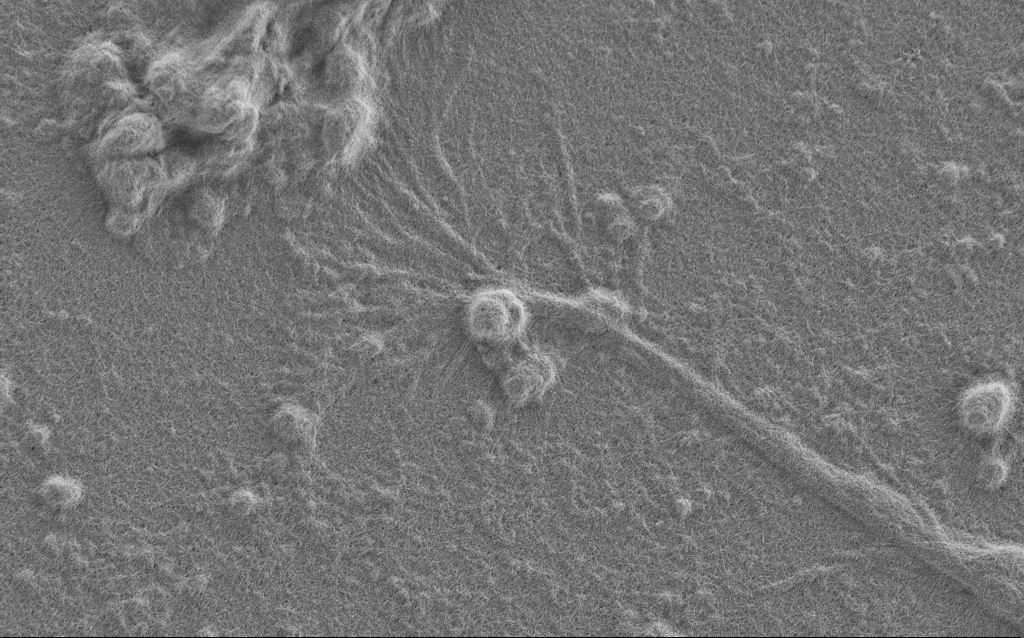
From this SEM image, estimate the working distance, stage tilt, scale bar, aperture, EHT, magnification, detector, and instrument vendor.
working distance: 6 mm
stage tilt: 0°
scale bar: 2000 nm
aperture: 30 µm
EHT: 1 kV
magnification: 7.5 K X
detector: SE2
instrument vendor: Zeiss